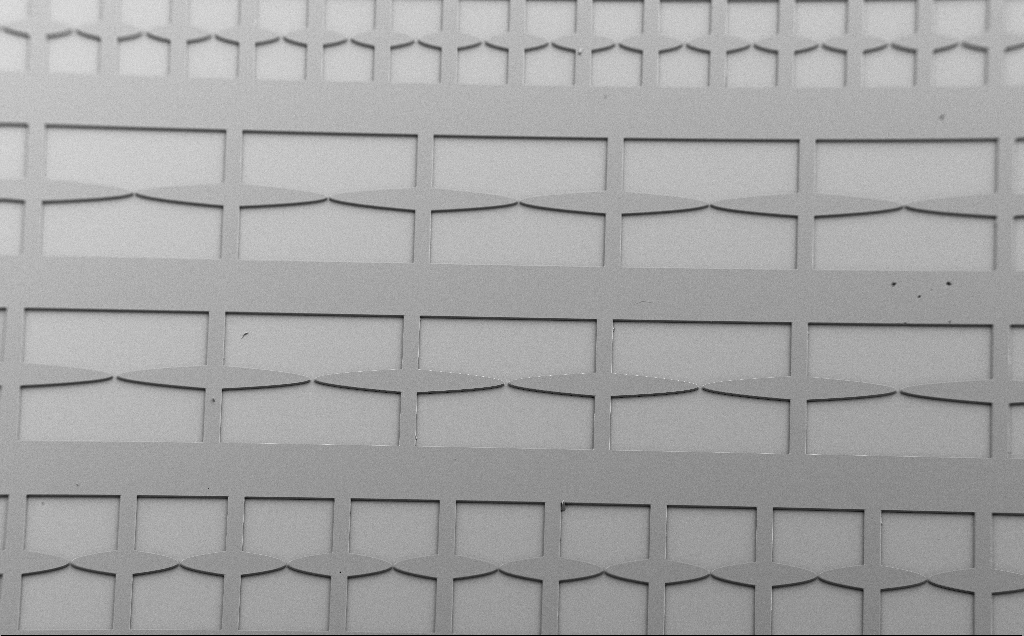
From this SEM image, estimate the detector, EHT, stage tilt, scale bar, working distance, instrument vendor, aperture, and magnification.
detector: SE2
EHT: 5 kV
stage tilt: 40°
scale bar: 200000 nm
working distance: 9 mm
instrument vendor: Zeiss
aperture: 30 µm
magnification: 0.122 K X